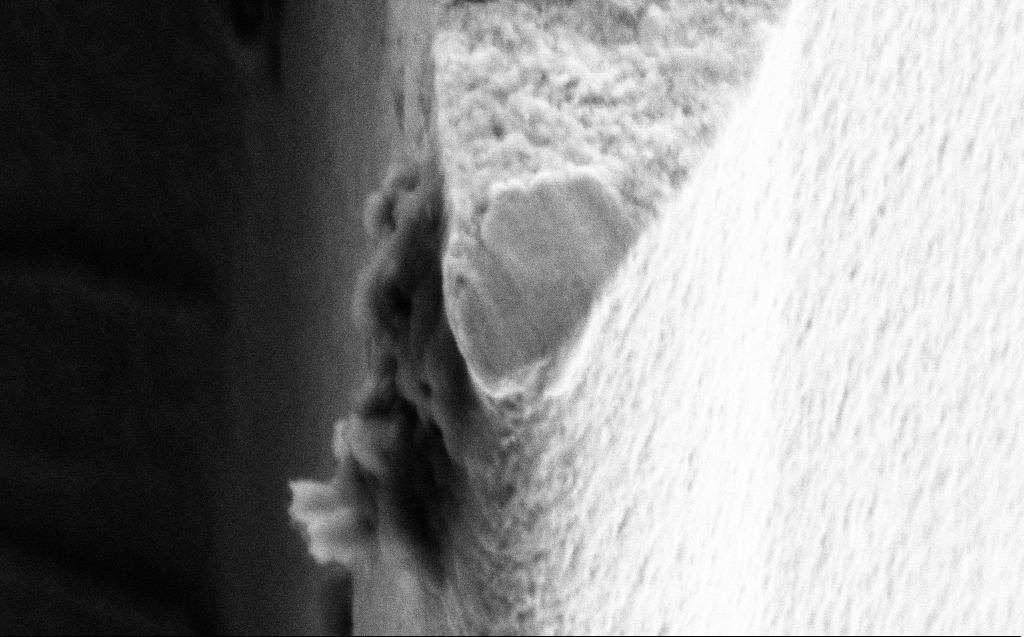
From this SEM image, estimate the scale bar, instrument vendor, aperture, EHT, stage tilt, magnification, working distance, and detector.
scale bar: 1000 nm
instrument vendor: Zeiss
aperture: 30 µm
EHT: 3 kV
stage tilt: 45°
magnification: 70.07 K X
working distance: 8 mm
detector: SE2